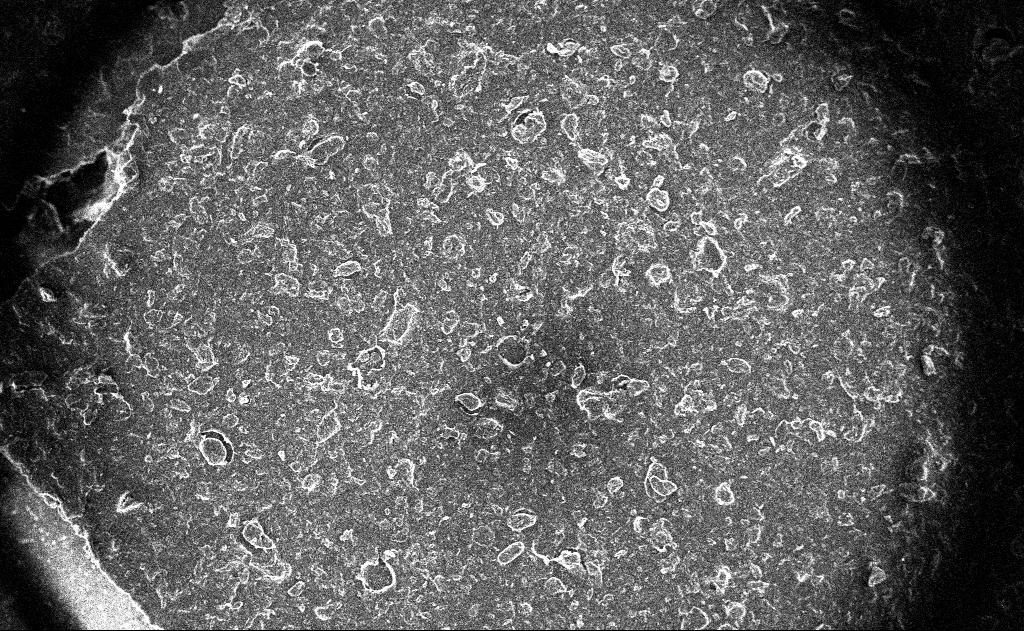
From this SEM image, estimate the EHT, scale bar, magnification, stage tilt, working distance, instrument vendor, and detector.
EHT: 10 kV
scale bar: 100000 nm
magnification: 0.134 K X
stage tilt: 0°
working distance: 3 mm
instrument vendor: Zeiss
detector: InLens